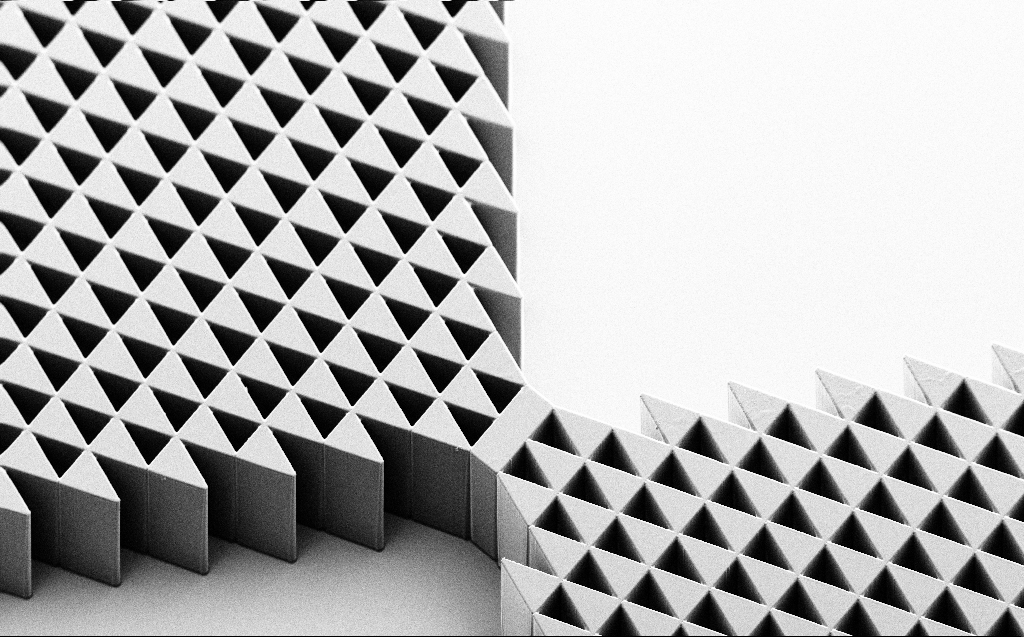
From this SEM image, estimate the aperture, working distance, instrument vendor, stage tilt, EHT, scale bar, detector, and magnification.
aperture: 30 µm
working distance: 11 mm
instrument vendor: Zeiss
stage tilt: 45°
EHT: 5 kV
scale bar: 200000 nm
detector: SE2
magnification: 0.264 K X